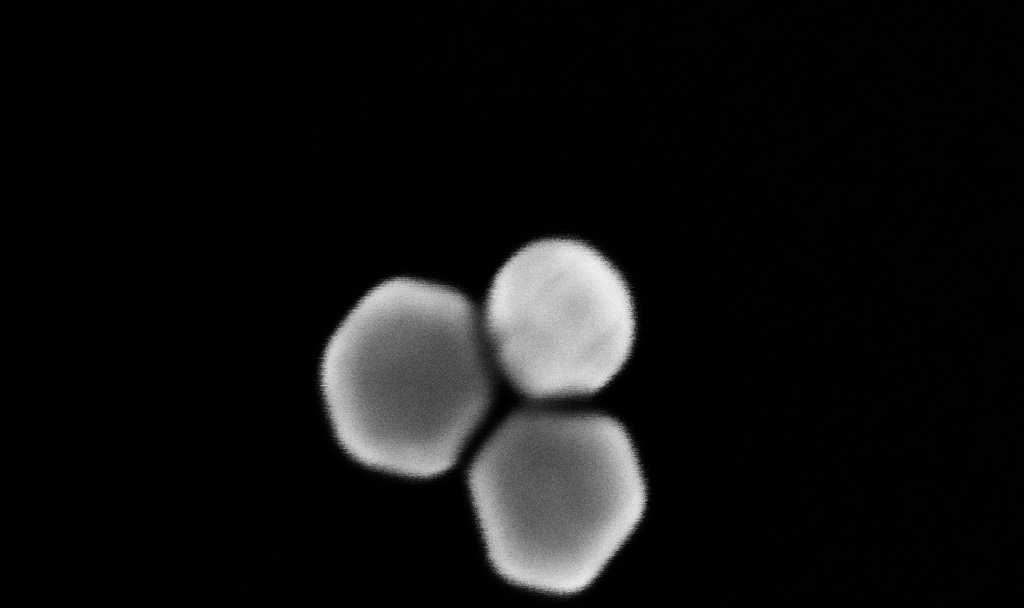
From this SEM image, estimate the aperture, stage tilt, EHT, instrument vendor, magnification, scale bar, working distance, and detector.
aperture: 30 µm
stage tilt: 0°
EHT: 10 kV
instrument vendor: Zeiss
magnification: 957.62 K X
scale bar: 20 nm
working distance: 3.4 mm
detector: InLens